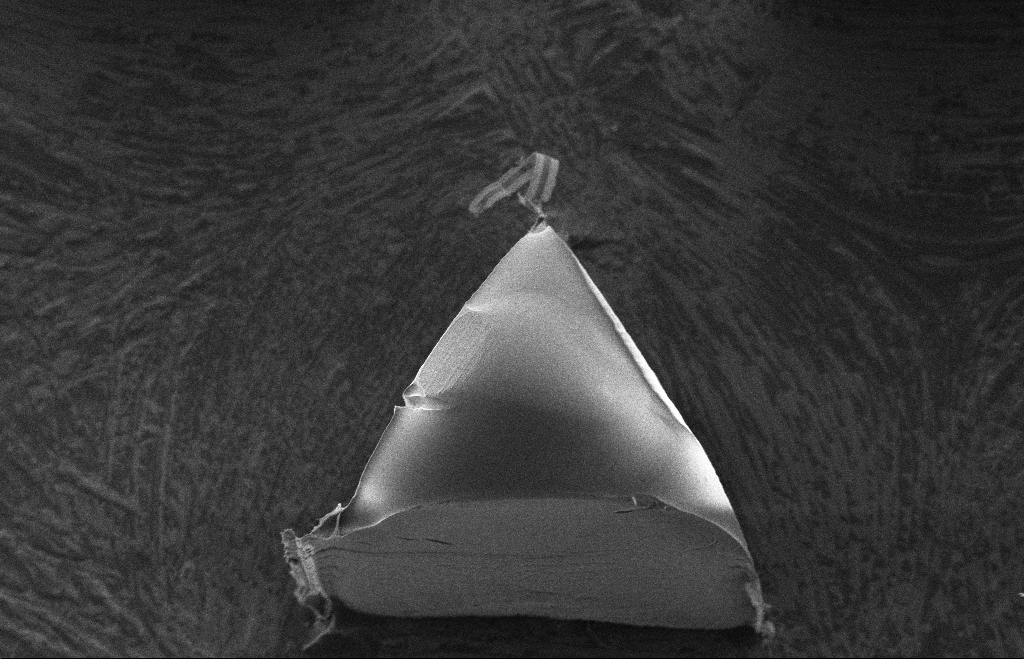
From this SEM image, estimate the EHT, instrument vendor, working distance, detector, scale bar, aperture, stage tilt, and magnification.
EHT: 5 kV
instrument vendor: Zeiss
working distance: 13 mm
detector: SE2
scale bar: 20000 nm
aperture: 30 µm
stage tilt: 14.7°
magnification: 0.619 K X